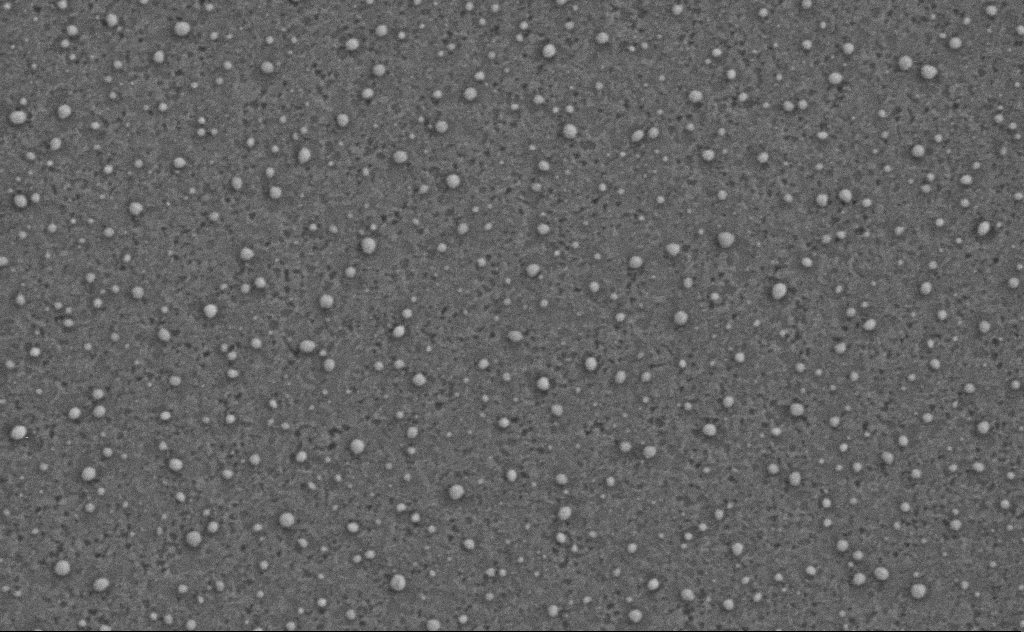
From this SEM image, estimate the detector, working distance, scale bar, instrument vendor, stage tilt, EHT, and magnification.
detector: SE2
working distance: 4 mm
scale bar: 200 nm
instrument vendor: Zeiss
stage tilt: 0°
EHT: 3 kV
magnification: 80 K X